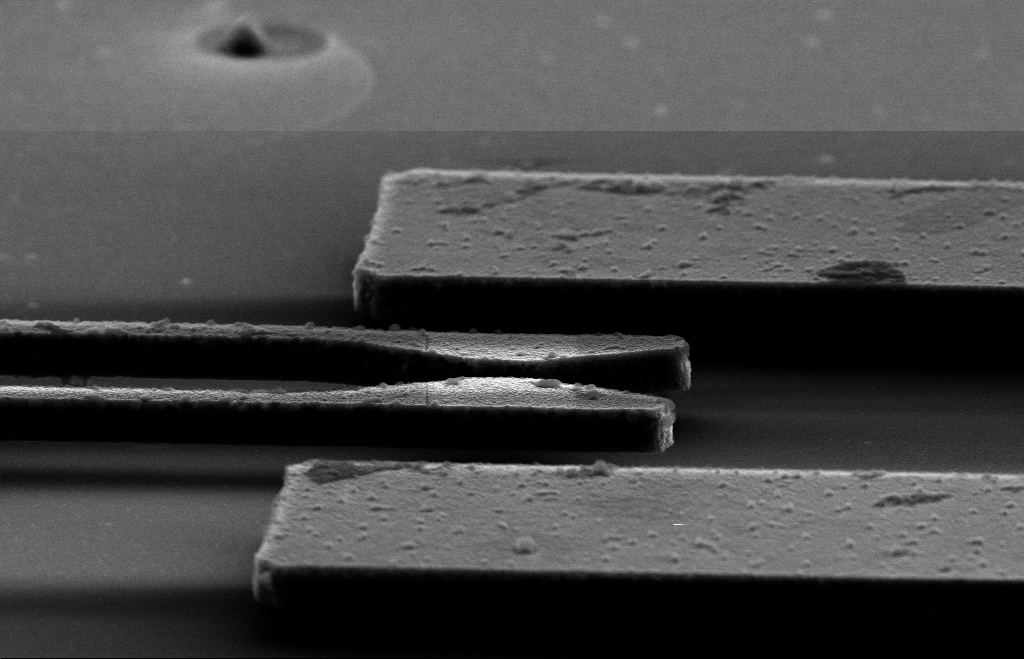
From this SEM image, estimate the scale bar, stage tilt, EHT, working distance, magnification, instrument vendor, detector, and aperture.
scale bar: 2000 nm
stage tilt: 70°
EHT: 10 kV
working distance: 11 mm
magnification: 6.84 K X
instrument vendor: Zeiss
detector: SE2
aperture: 30 µm